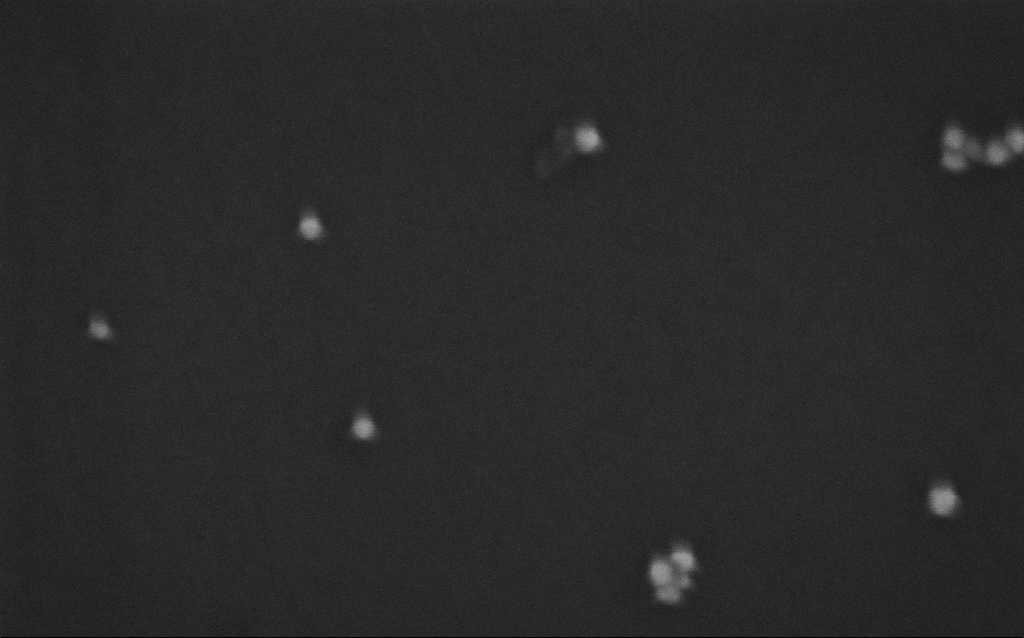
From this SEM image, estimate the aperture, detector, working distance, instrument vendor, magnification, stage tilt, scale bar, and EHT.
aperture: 30 µm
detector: InLens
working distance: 7 mm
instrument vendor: Zeiss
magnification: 500 K X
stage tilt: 0°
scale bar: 100 nm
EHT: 10 kV